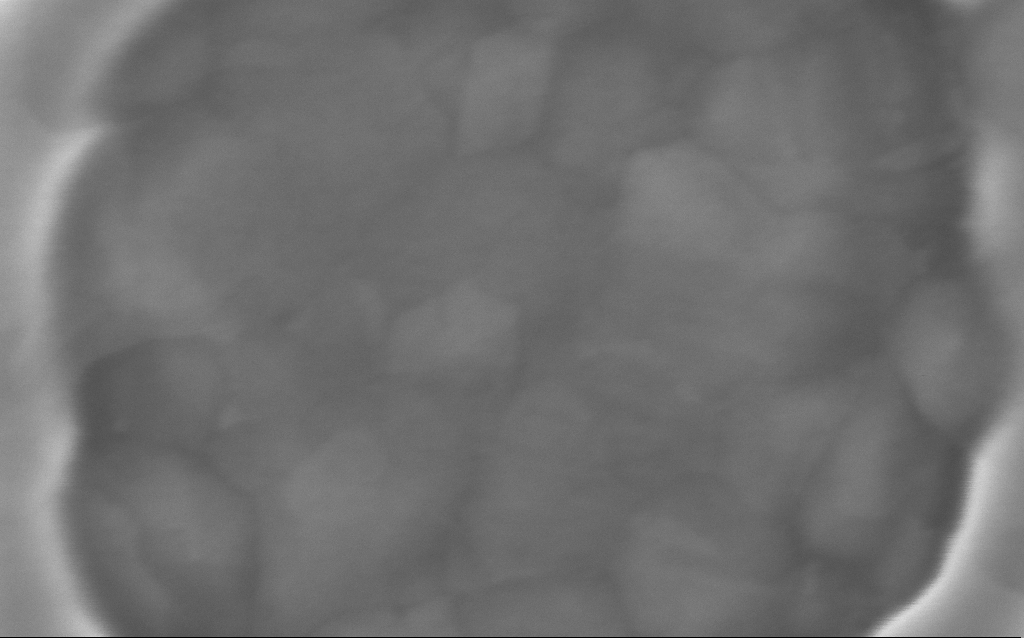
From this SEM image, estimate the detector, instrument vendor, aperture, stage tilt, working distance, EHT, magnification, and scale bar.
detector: InLens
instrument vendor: Zeiss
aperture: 30 µm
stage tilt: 0°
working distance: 5 mm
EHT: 5 kV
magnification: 515.1 K X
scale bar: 100 nm